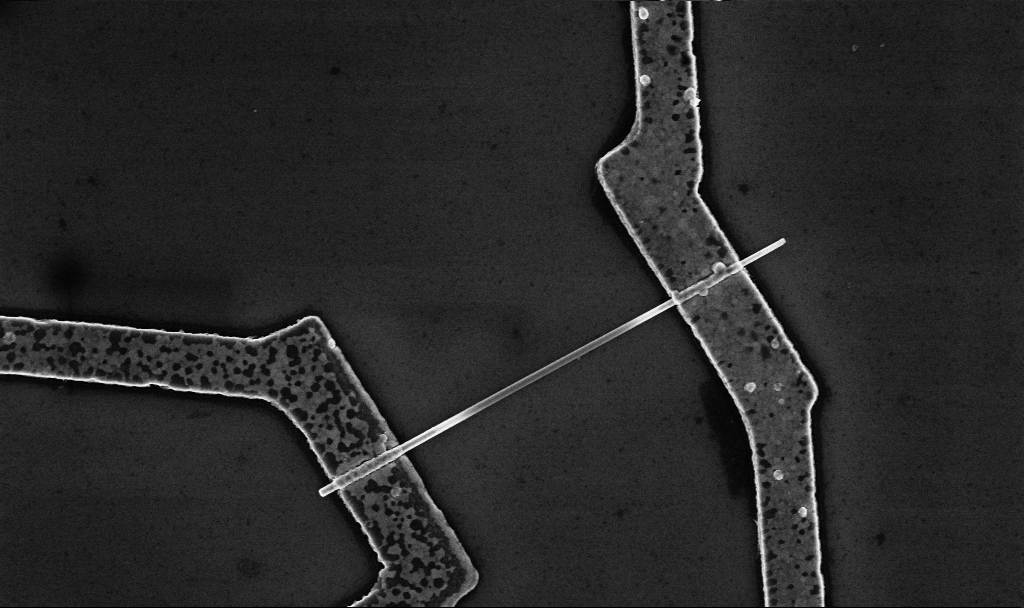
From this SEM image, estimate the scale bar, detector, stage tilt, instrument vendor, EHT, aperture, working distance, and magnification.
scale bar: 1000 nm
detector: InLens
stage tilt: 0°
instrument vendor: Zeiss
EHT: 5 kV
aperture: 30 µm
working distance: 8.7 mm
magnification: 30 K X